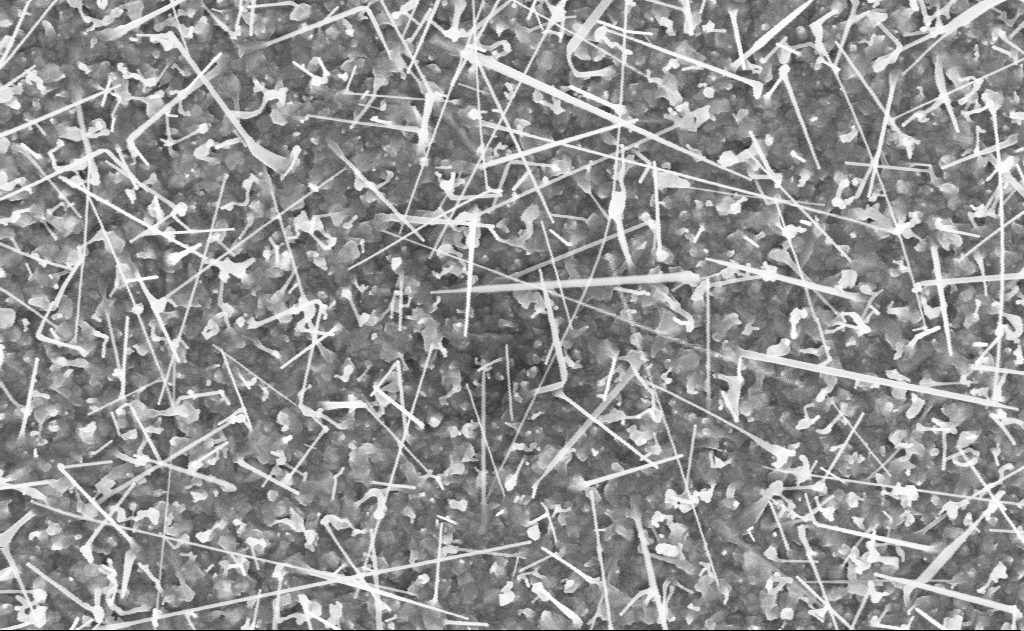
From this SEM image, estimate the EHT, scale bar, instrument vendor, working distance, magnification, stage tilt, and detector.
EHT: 10 kV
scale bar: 1000 nm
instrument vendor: Zeiss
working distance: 20 mm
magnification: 40 K X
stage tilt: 0°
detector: InLens